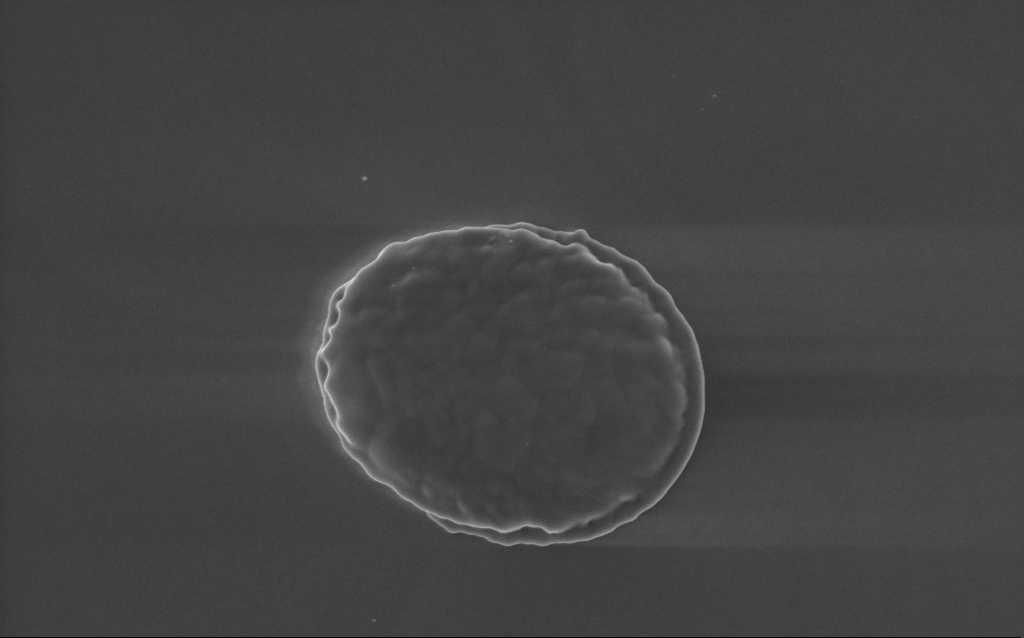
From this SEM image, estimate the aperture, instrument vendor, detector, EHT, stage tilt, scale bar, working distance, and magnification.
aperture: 30 µm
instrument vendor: Zeiss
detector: InLens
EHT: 5 kV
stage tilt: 0°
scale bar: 1000 nm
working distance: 3 mm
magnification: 44 K X